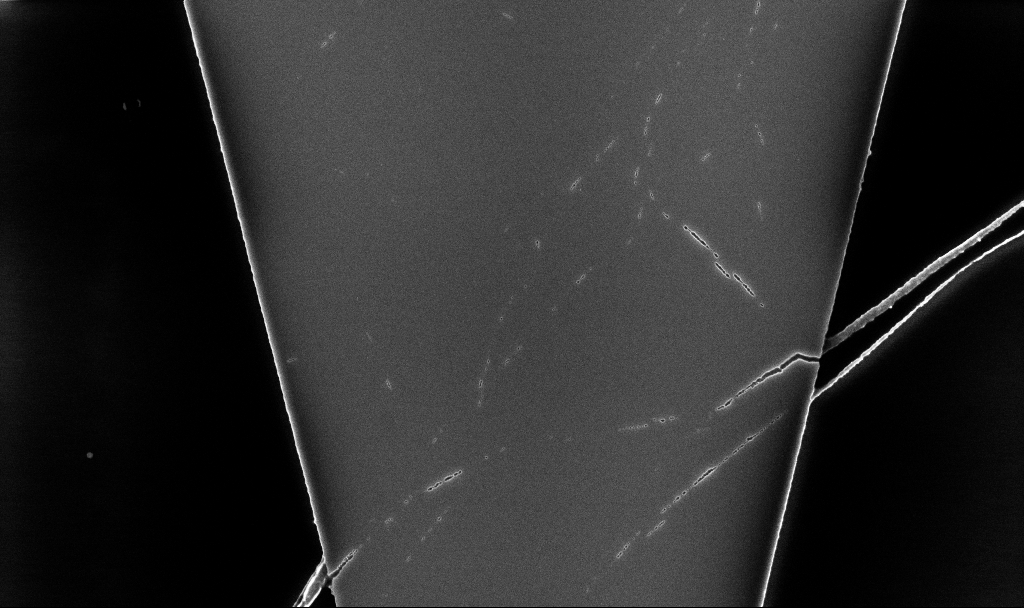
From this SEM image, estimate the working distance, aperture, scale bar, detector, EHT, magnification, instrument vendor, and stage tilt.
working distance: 5.2 mm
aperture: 30 µm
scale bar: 2000 nm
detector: InLens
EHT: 5 kV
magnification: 14.12 K X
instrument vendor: Zeiss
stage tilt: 0°